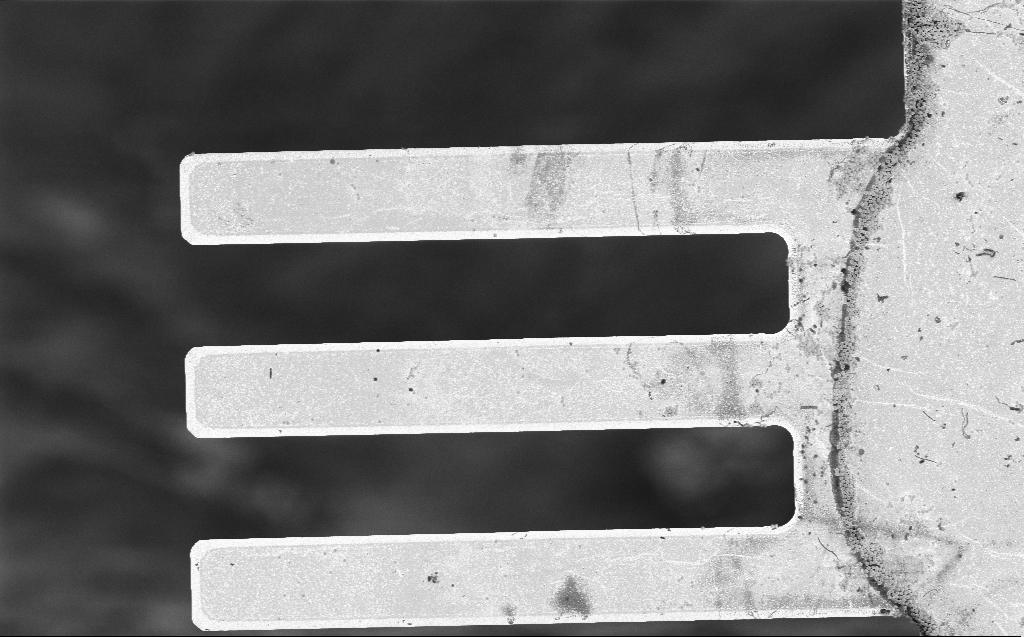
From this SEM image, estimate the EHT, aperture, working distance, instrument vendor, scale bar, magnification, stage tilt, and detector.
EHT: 3 kV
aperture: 30 µm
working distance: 7 mm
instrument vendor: Zeiss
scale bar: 20000 nm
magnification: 1.78 K X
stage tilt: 0°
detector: InLens